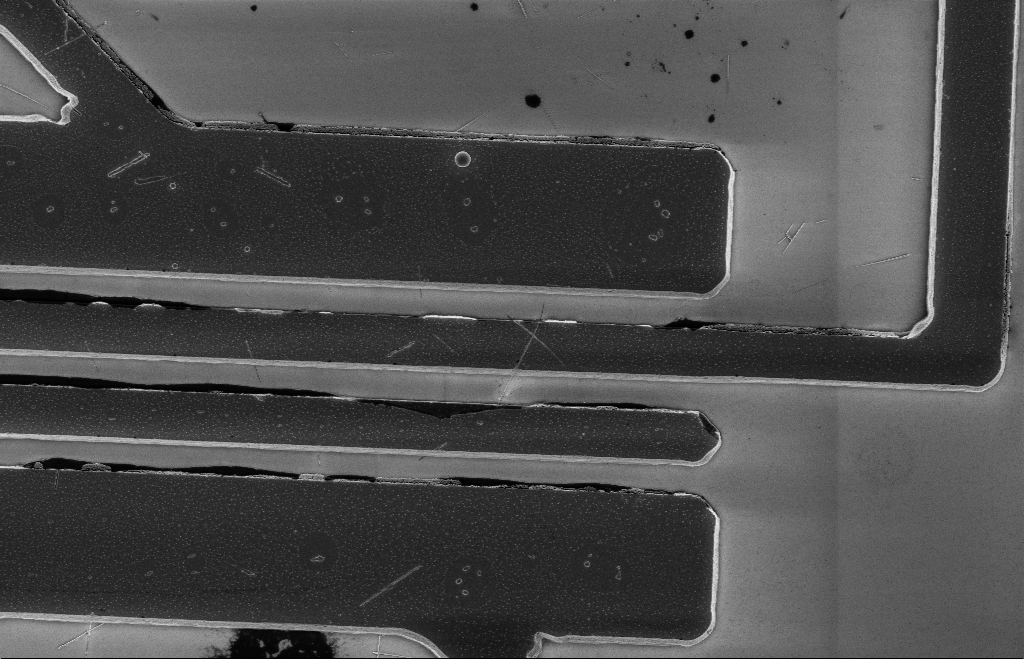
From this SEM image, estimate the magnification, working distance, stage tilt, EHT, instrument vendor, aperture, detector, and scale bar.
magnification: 5.12 K X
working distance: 12 mm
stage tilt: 0°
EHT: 5 kV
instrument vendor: Zeiss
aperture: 20 µm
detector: InLens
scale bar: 2000 nm